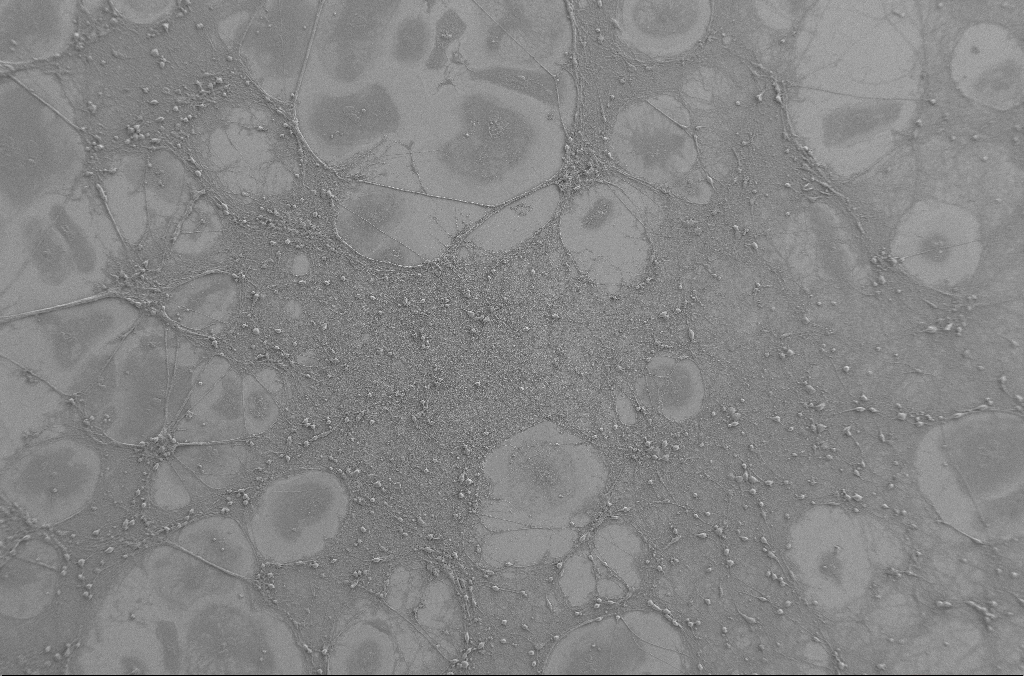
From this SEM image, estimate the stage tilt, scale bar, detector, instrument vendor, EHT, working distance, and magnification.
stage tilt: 0°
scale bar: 100000 nm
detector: SE2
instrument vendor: Zeiss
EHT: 2 kV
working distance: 4 mm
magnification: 0.25 K X